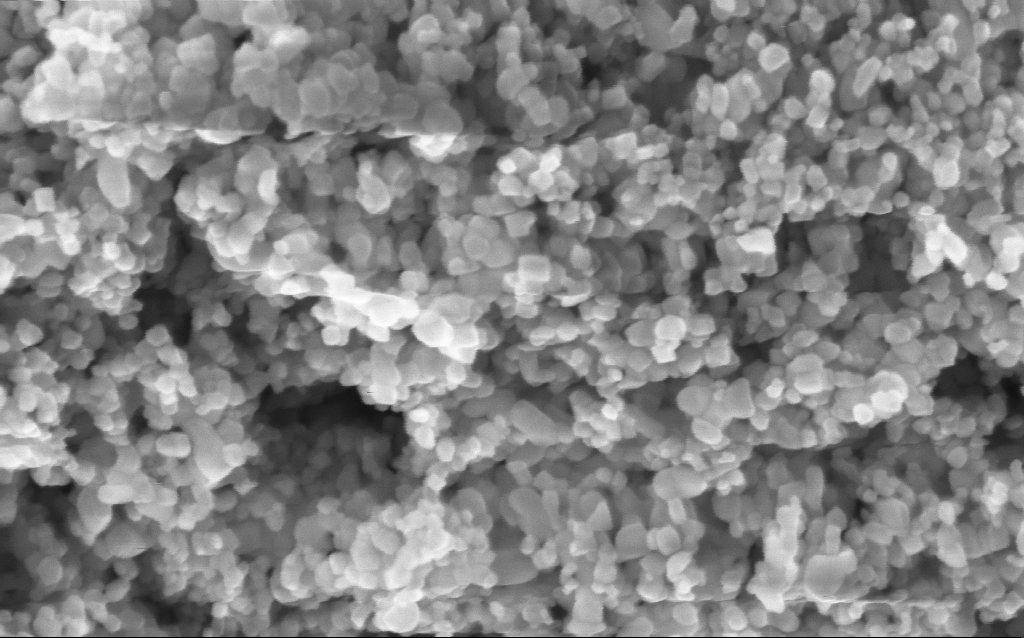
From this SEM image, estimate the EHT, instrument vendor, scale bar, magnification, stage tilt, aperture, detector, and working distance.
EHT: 5 kV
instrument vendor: Zeiss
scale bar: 200 nm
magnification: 294.05 K X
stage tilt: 0°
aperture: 30 µm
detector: InLens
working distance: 4.4 mm